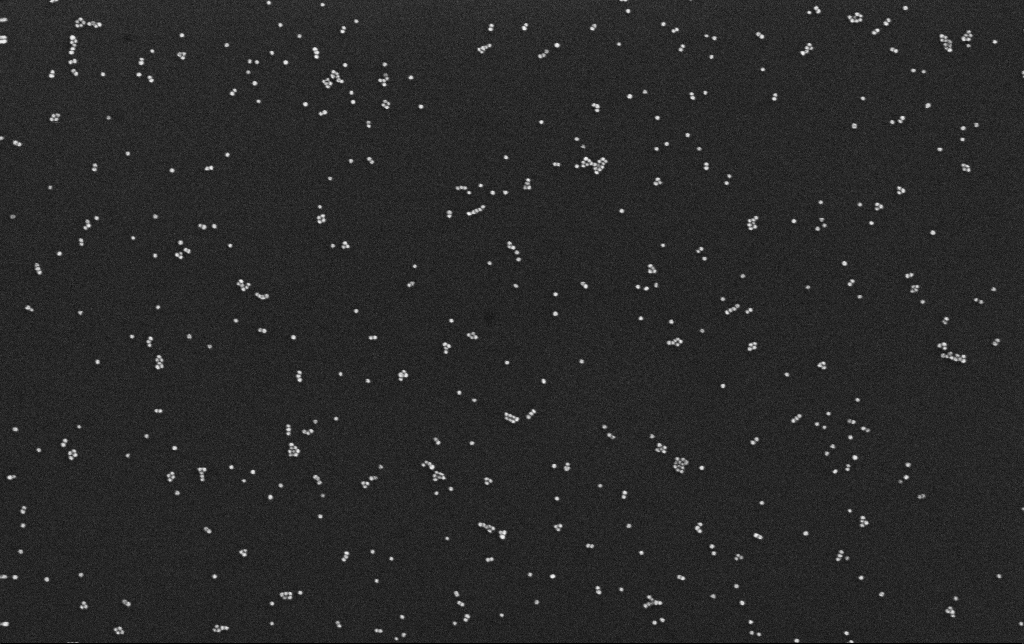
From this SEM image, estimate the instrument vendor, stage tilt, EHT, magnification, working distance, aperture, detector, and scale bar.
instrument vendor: Zeiss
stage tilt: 0°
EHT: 10 kV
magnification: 100 K X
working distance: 3.1 mm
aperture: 30 µm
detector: InLens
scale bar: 200 nm